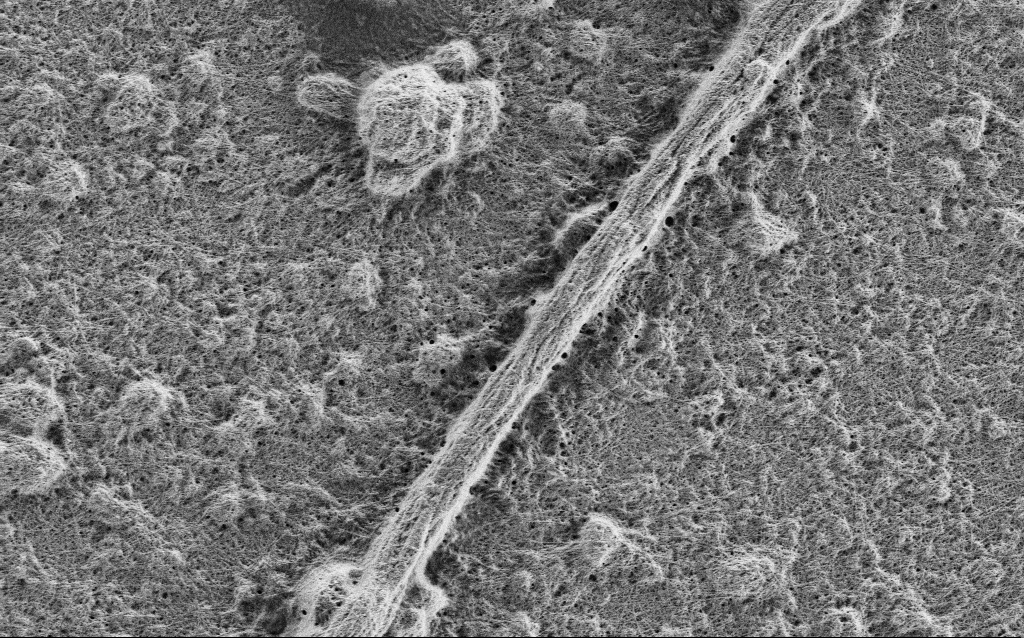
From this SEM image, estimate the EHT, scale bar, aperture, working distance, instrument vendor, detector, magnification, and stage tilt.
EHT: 1 kV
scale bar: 2000 nm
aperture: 30 µm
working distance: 4 mm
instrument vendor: Zeiss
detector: SE2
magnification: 10 K X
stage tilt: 0°